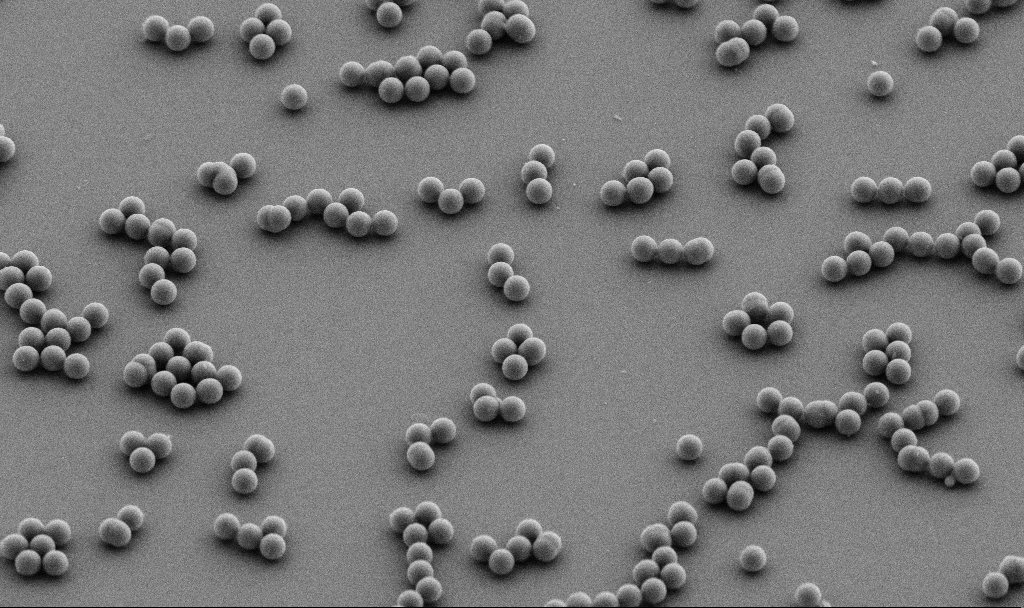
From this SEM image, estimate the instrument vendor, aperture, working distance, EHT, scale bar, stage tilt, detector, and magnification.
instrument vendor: Zeiss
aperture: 30 µm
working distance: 7.7 mm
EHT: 5 kV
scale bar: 2000 nm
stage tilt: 45°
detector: SE2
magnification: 7.43 K X